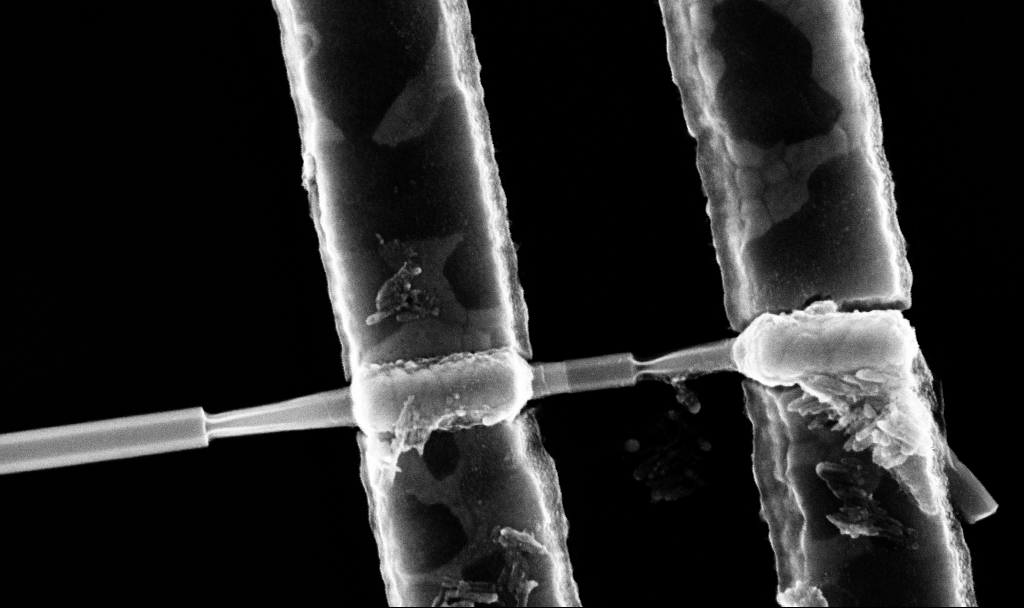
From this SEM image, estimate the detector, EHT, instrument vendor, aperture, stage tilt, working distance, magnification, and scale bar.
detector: InLens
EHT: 10 kV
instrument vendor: Zeiss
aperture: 30 µm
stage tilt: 0°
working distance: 7.7 mm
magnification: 113.38 K X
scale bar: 200 nm